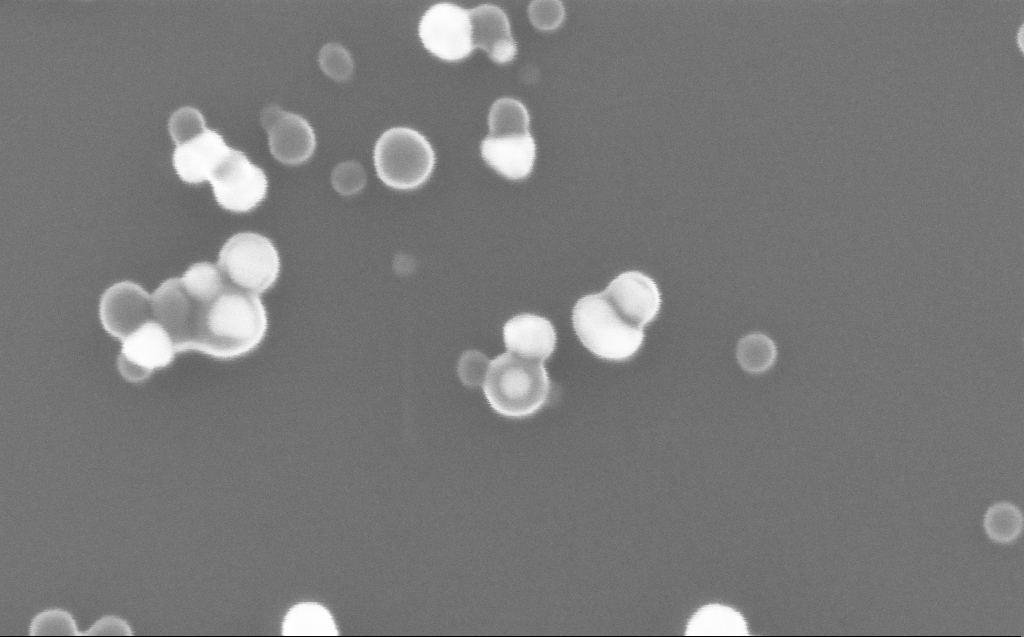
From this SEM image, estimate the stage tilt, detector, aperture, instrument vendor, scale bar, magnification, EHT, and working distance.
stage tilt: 0°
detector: InLens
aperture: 30 µm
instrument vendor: Zeiss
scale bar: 100 nm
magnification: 400 K X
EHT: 10 kV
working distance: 3 mm